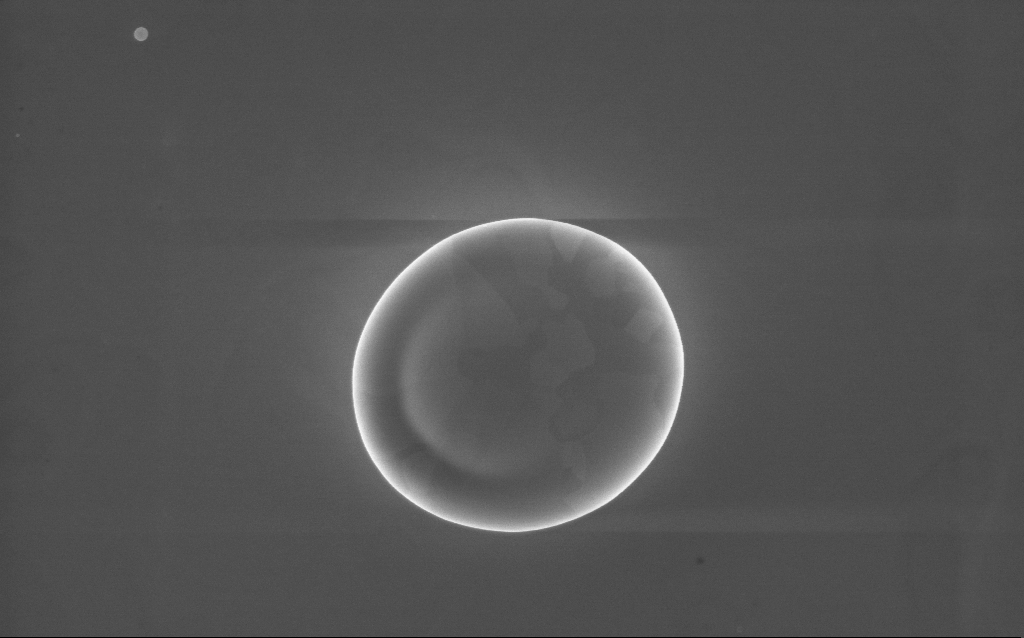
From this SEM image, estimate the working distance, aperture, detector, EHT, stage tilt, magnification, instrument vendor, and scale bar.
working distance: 2 mm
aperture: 30 µm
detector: InLens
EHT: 10 kV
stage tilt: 0°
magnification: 36 K X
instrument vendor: Zeiss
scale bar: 1000 nm